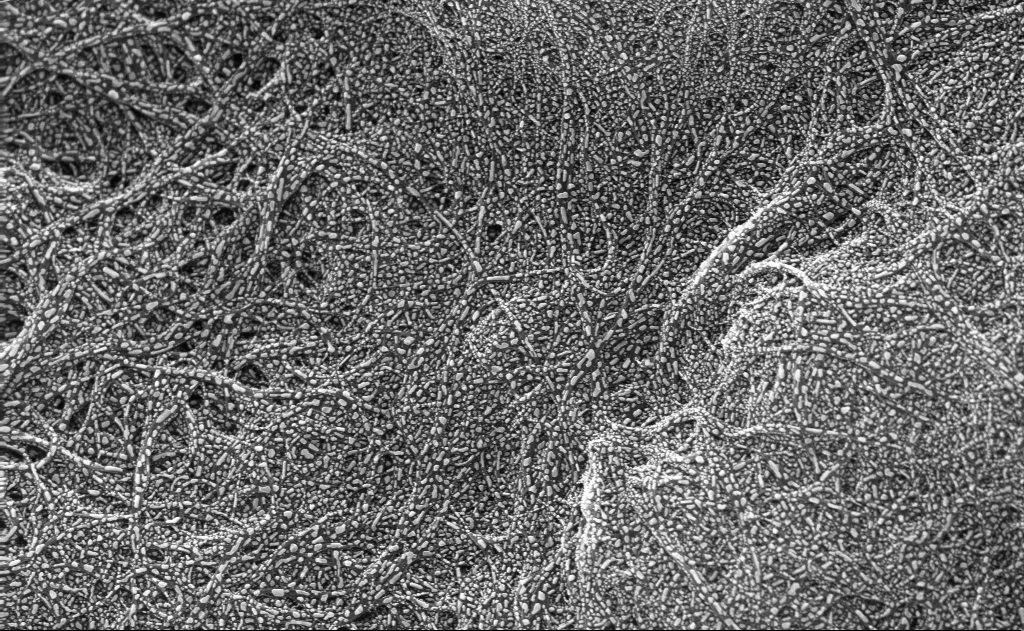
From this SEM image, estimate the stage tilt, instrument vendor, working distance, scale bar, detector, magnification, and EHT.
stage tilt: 0°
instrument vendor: Zeiss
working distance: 3 mm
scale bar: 200 nm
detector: InLens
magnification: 79.56 K X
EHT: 10 kV